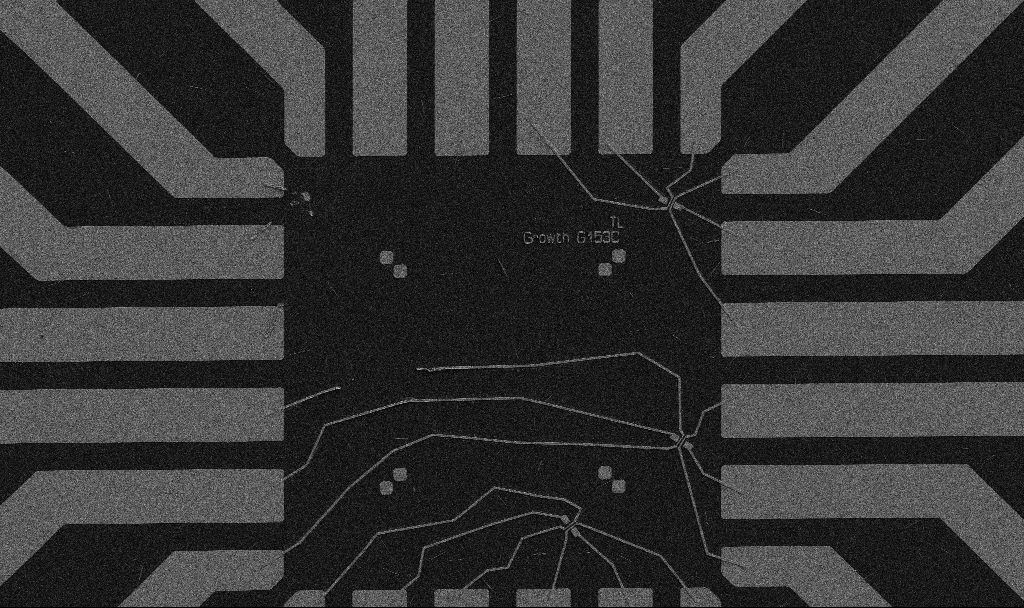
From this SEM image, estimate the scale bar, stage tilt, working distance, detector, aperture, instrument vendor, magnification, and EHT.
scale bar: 20000 nm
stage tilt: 0°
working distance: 10.7 mm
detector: SE2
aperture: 30 µm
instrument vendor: Zeiss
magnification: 1 K X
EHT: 5 kV